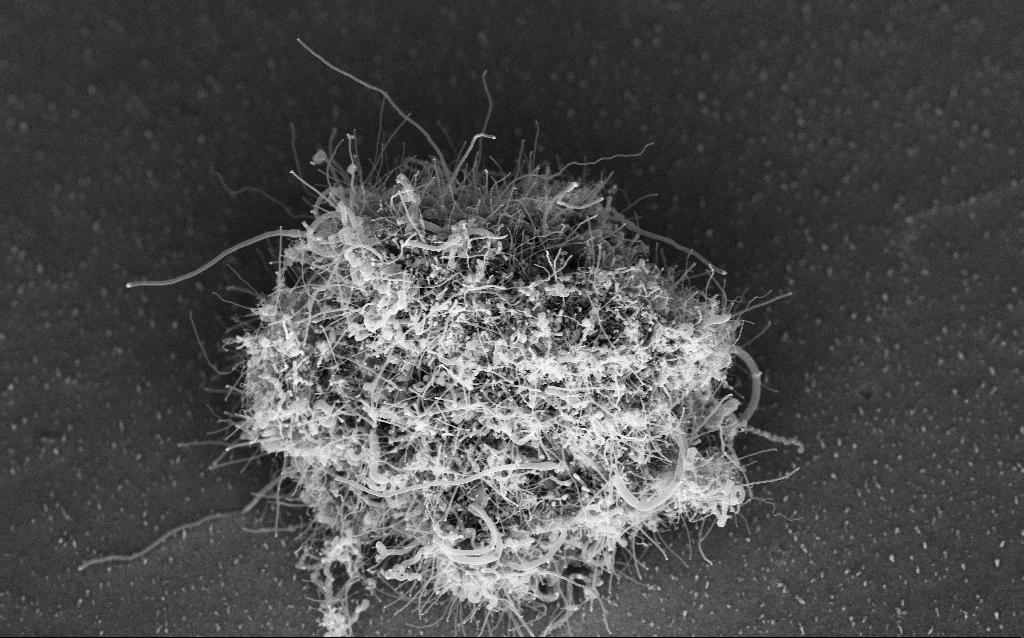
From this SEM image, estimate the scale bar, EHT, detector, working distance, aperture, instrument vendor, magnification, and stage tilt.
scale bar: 1000 nm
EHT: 5 kV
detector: InLens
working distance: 6.6 mm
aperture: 30 µm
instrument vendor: Zeiss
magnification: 13.18 K X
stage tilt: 45°